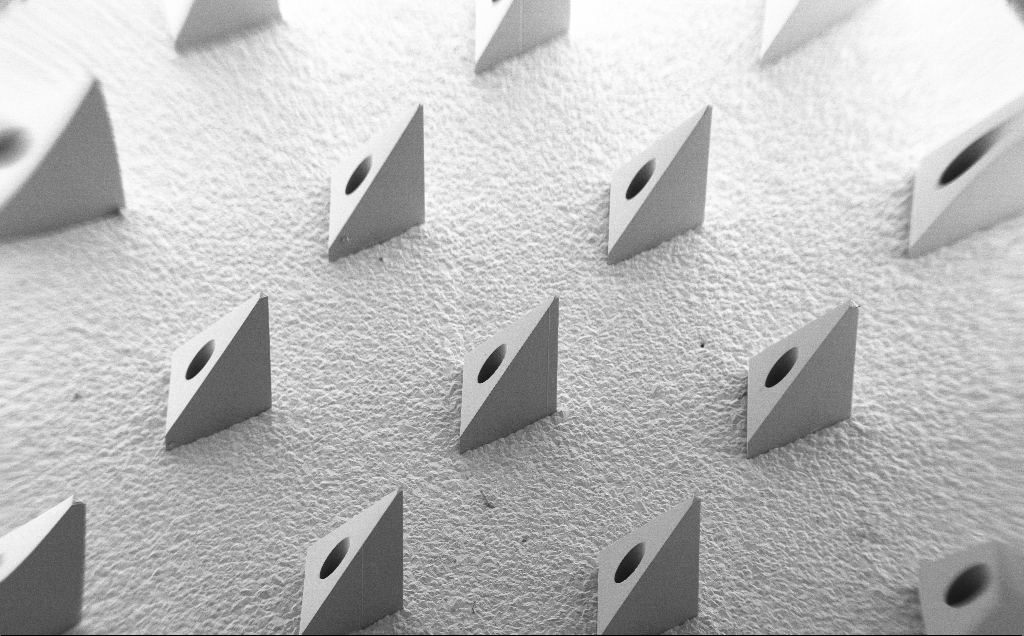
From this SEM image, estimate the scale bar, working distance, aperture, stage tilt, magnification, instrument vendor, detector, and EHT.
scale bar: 1e+06 nm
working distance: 9 mm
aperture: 30 µm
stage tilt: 40°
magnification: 0.067 K X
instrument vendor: Zeiss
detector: SE2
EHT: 5 kV